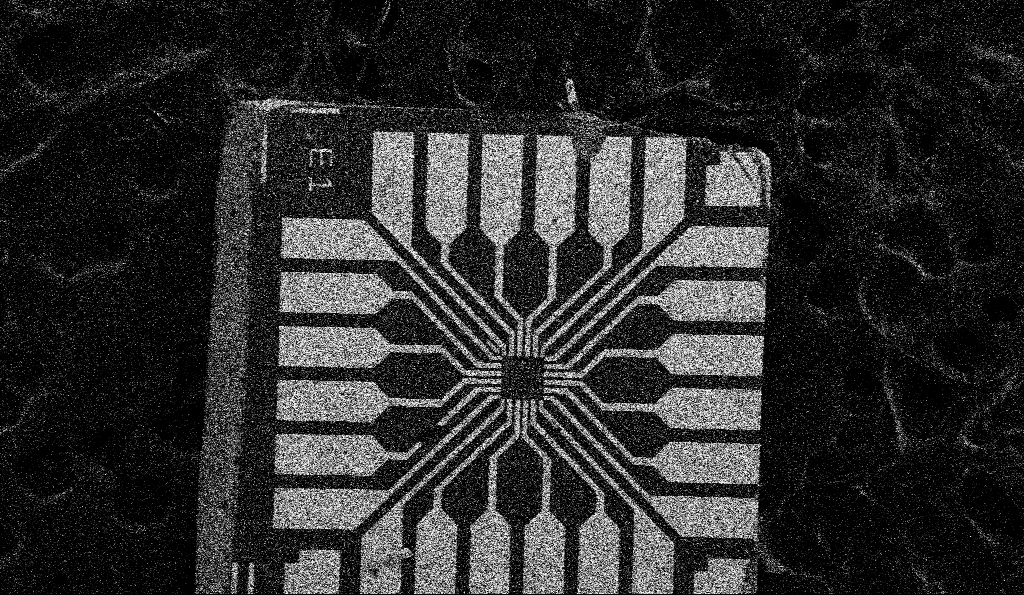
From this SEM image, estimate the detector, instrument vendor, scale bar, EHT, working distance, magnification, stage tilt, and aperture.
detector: SE2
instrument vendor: Zeiss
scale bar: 200000 nm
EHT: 5 kV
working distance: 8.5 mm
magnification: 0.1 K X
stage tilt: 0°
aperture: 30 µm